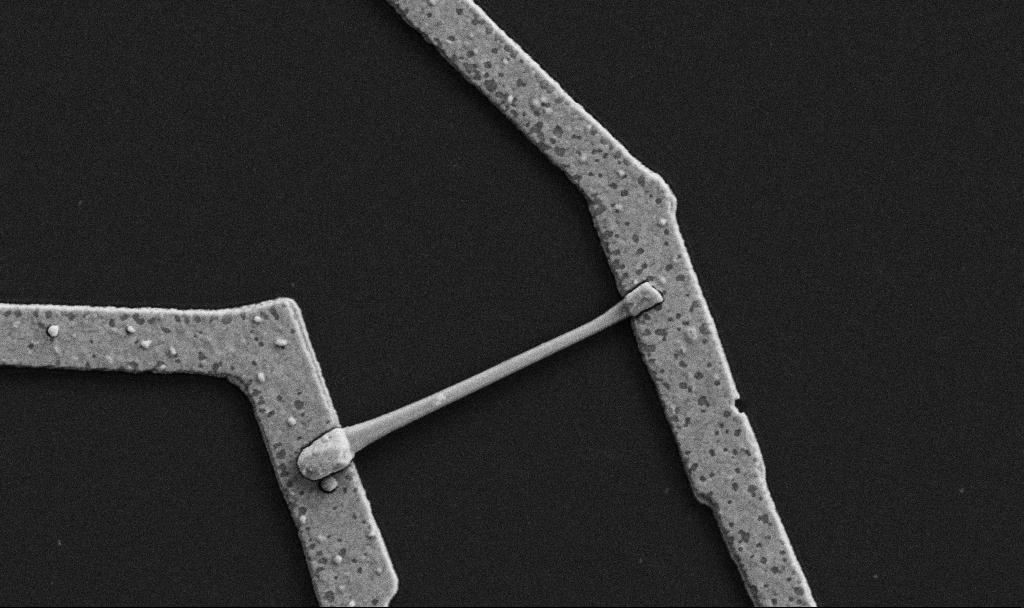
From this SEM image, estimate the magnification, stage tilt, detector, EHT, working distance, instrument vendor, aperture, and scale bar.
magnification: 30 K X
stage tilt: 0°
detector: SE2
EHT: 5 kV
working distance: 8.7 mm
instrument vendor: Zeiss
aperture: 30 µm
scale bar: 1000 nm